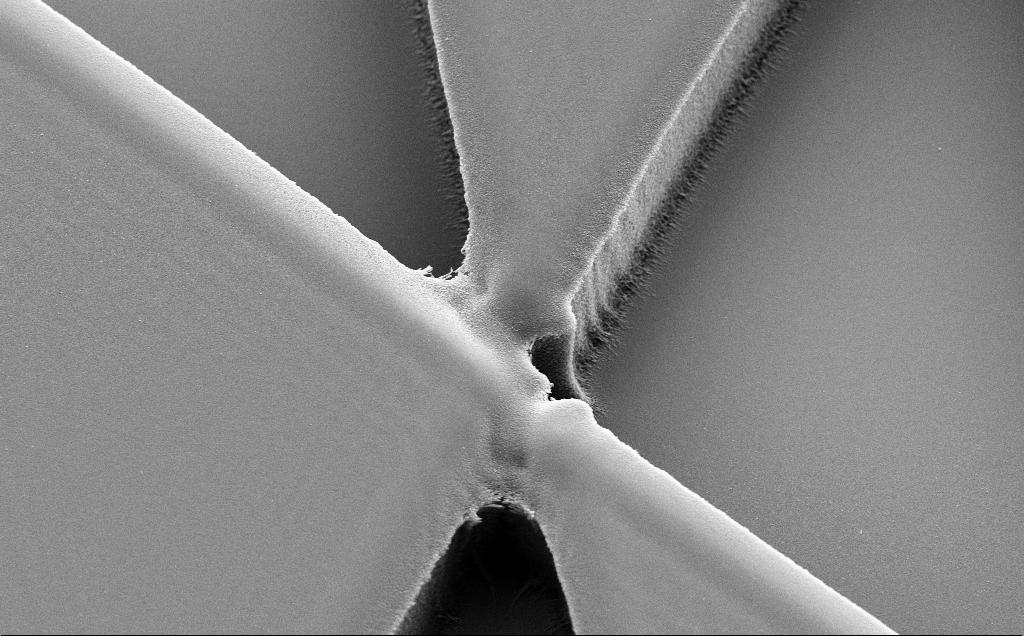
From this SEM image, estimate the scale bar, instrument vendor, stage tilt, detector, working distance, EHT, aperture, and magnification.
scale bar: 10000 nm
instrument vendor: Zeiss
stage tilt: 30°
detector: SE2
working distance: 10 mm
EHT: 5 kV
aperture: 30 µm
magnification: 6.85 K X